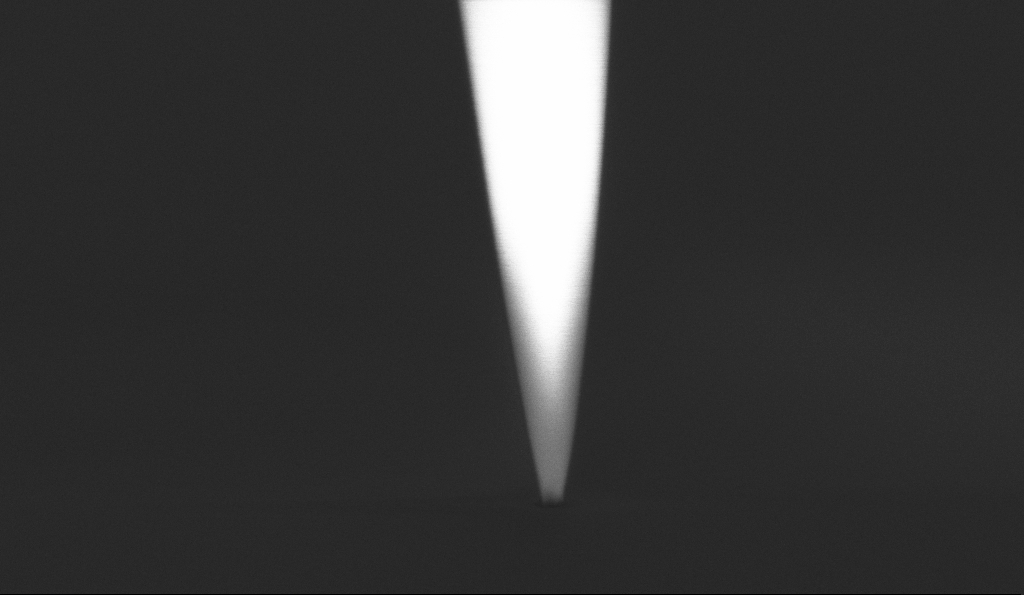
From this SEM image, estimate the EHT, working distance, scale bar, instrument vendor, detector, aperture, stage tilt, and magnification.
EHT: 1 kV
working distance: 5.6 mm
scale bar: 200 nm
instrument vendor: Zeiss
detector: InLens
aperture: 30 µm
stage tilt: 0°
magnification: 100 K X